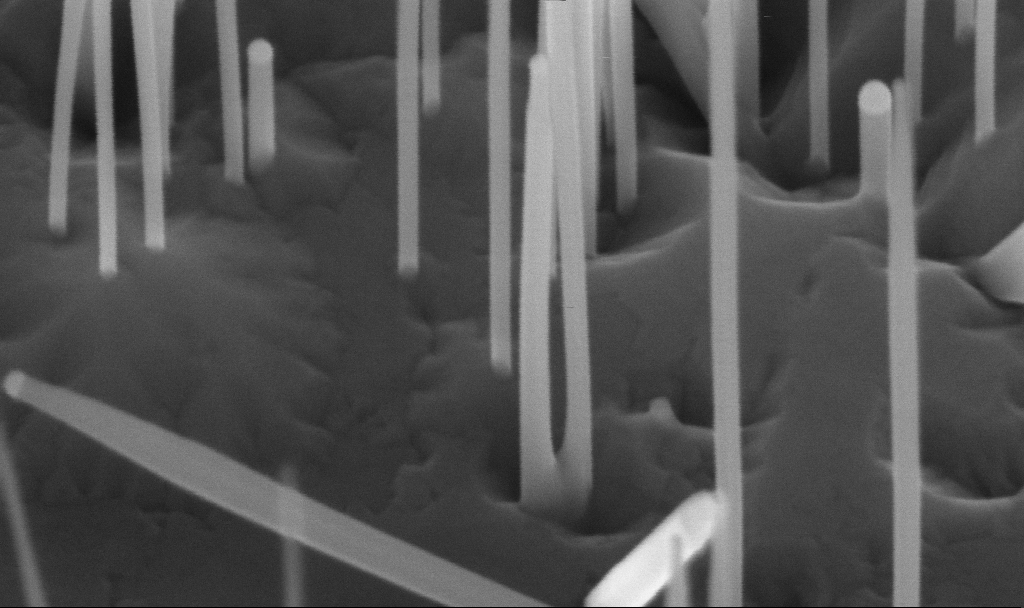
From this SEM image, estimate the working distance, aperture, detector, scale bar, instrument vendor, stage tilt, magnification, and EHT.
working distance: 5.6 mm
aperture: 30 µm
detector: InLens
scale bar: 200 nm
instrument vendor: Zeiss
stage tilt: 45°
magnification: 324.89 K X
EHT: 10 kV